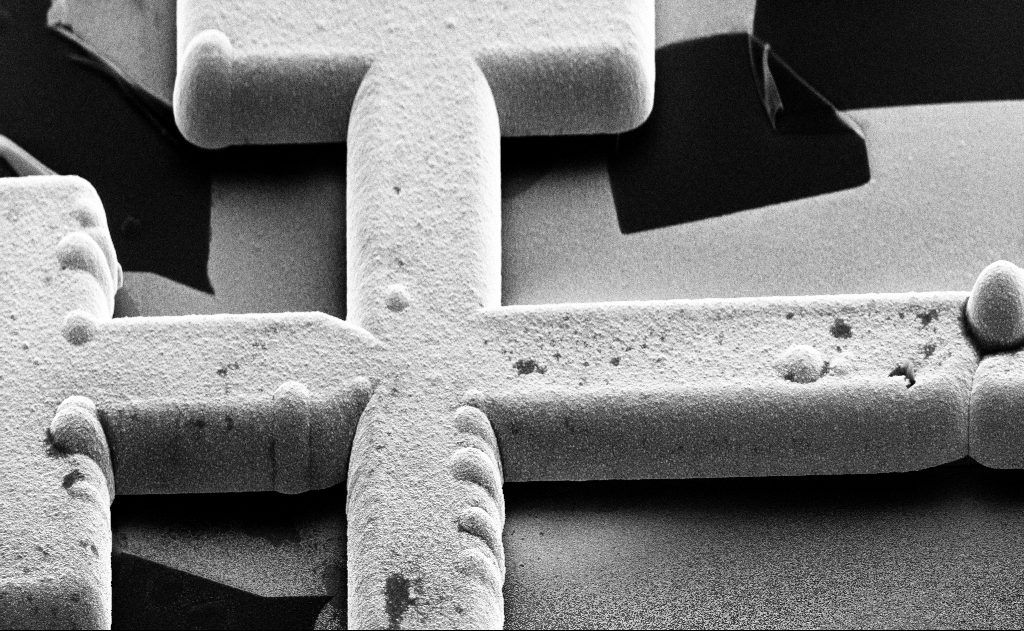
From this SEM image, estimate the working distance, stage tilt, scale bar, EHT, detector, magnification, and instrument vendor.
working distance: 13 mm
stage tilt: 60°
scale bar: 20000 nm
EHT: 10 kV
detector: SE2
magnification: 2.98 K X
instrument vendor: Zeiss